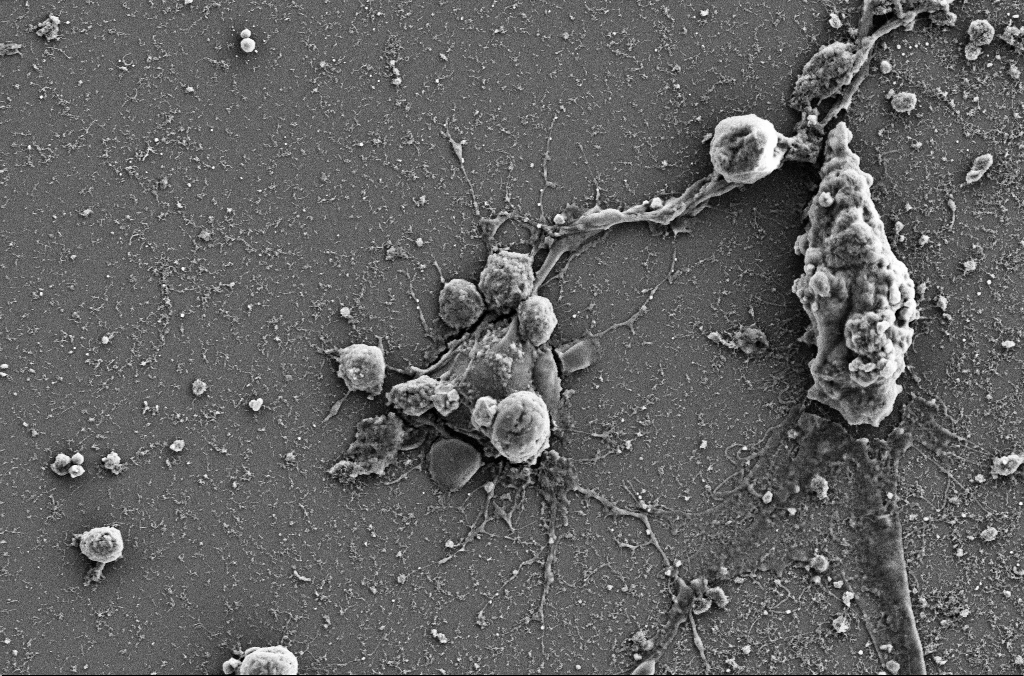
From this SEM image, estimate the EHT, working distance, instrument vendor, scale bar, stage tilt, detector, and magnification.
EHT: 5 kV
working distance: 4 mm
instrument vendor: Zeiss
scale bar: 10000 nm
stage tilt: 0°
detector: SE2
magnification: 4 K X